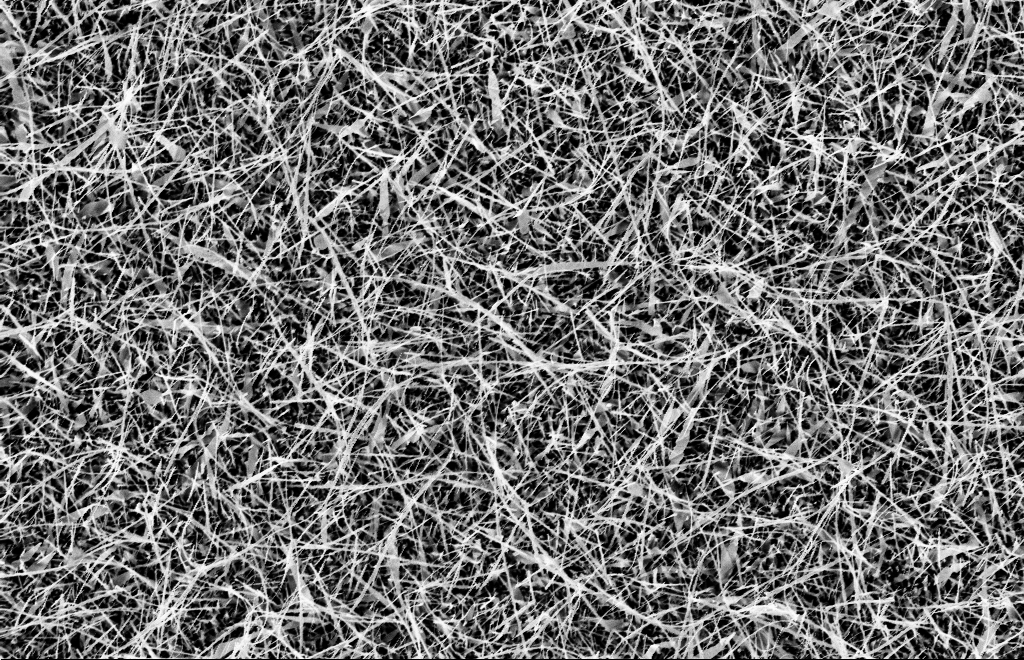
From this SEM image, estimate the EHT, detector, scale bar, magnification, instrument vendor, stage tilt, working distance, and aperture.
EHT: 10 kV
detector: InLens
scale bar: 2000 nm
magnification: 5 K X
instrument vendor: Zeiss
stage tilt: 0°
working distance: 9 mm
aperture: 20 µm